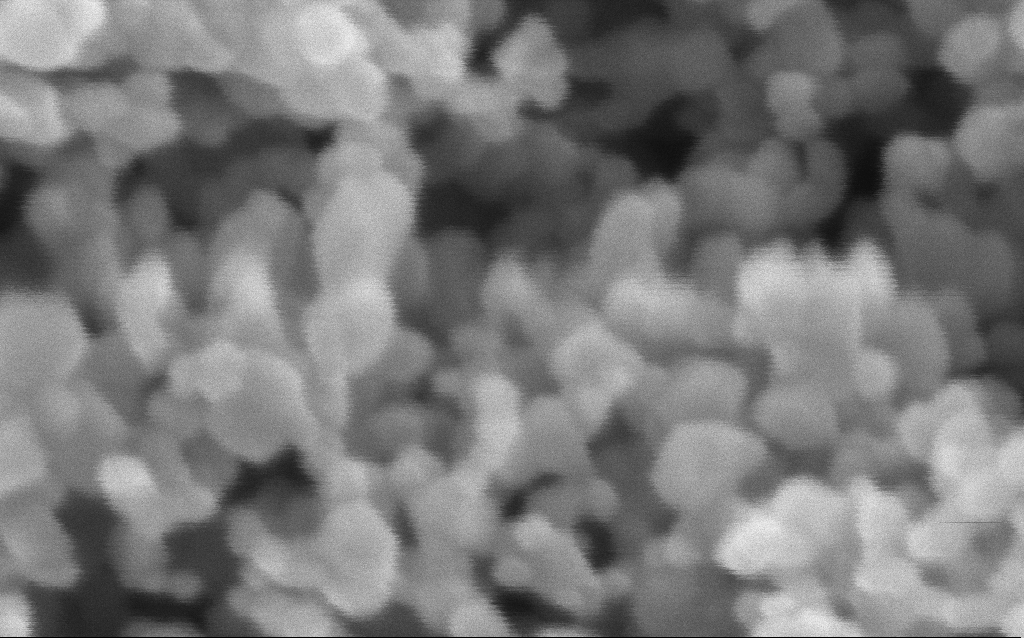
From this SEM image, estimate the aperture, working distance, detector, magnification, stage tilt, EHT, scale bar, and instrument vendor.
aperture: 30 µm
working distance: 7.5 mm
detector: InLens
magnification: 716 K X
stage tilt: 0°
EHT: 3 kV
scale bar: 100 nm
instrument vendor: Zeiss